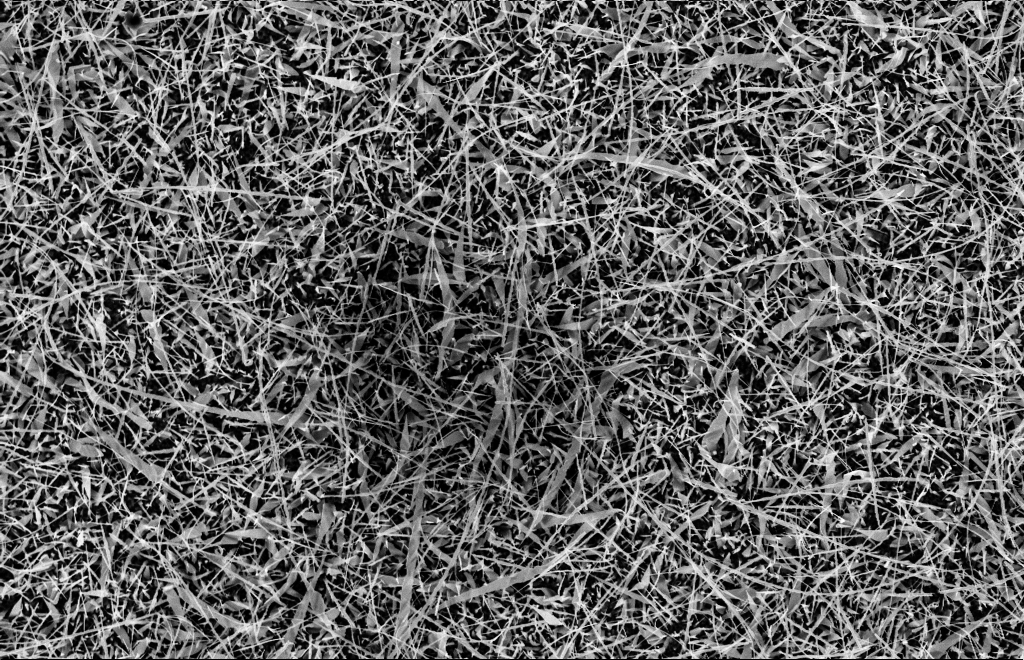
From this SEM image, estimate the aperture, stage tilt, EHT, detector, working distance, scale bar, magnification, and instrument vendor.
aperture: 30 µm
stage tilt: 0°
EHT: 10 kV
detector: InLens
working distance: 15 mm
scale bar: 2000 nm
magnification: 5 K X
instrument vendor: Zeiss